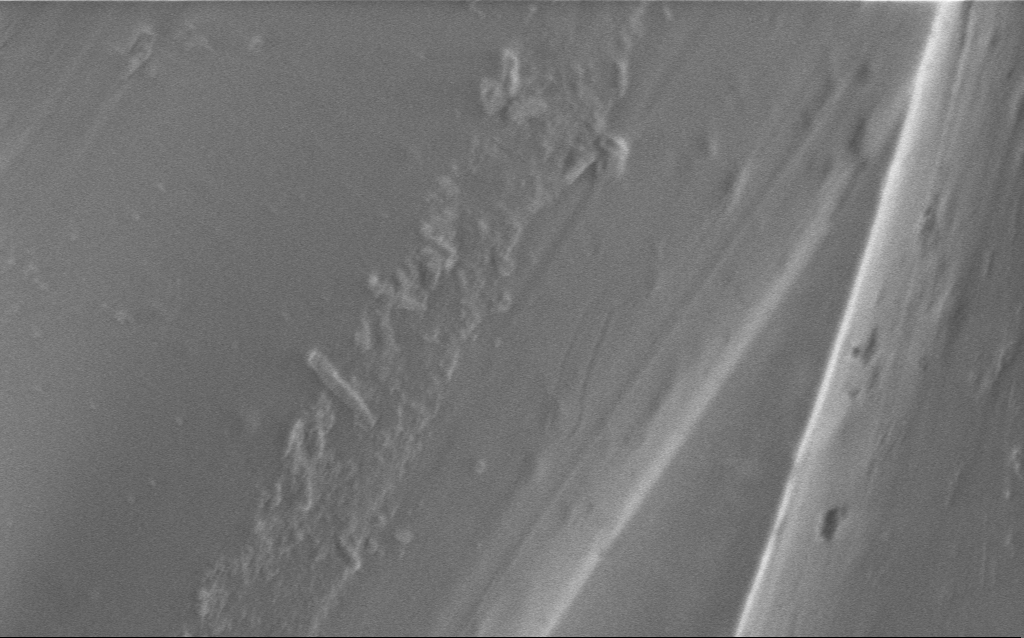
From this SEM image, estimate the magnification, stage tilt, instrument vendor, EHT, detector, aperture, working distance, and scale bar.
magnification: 14.13 K X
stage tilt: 0°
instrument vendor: Zeiss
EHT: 1 kV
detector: InLens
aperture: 30 µm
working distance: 4 mm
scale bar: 2000 nm